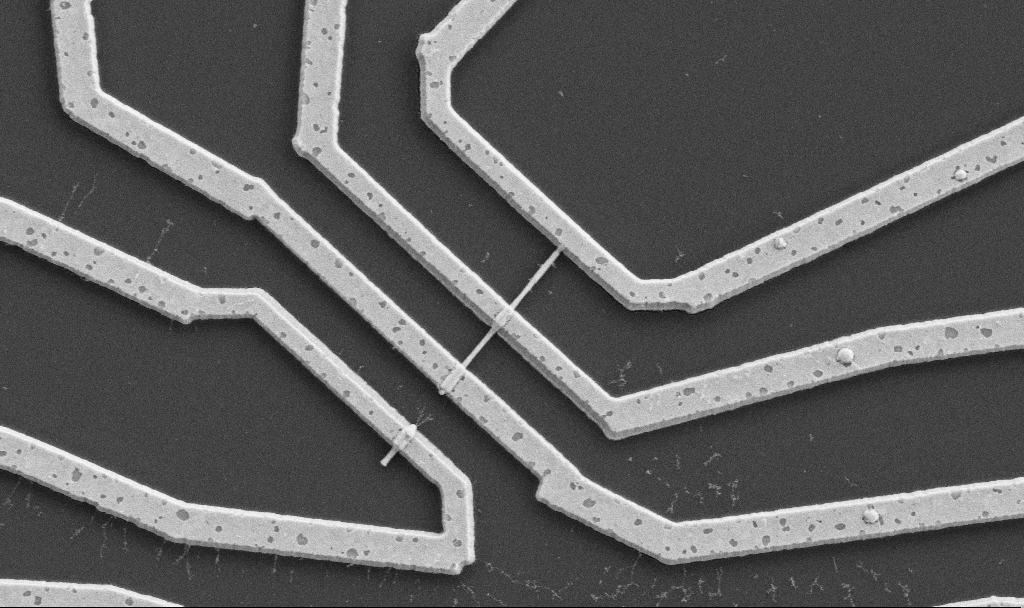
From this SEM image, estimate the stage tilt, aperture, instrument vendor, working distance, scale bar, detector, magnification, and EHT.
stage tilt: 0°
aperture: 30 µm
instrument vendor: Zeiss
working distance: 10.7 mm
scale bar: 1000 nm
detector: SE2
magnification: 20 K X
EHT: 5 kV